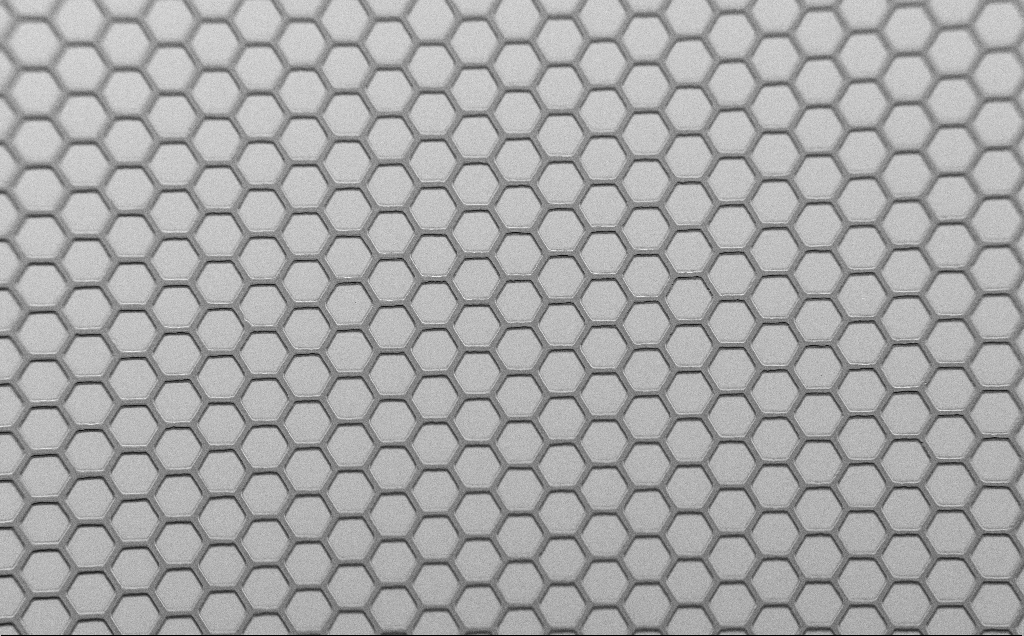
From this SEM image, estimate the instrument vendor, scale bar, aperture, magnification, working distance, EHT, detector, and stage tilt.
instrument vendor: Zeiss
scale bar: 200000 nm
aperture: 30 µm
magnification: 0.3 K X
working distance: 7 mm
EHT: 1.5 kV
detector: SE2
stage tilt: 45°